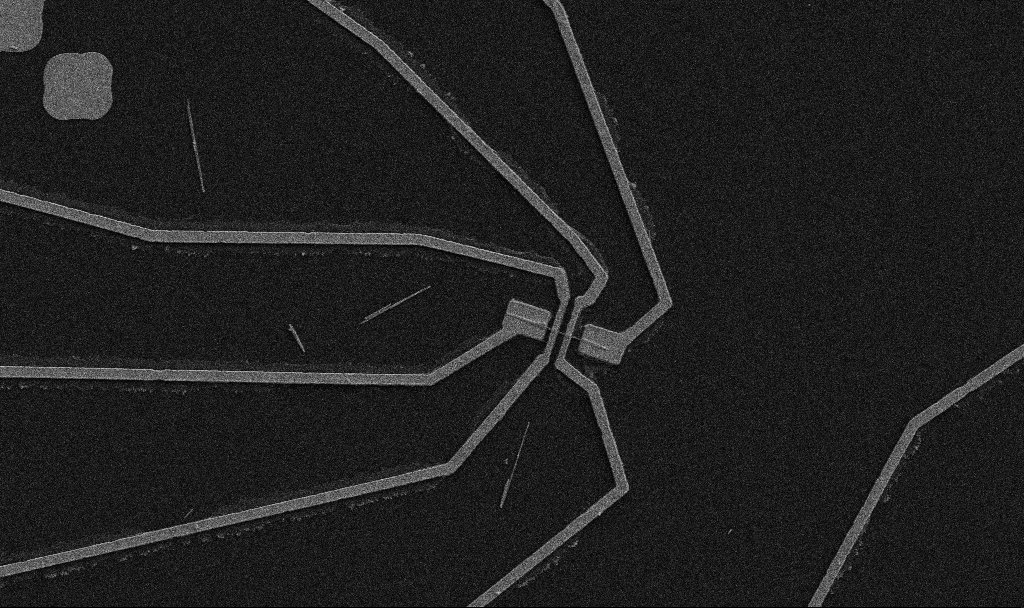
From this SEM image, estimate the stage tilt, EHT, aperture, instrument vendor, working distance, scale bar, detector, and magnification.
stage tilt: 0°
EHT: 5 kV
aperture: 30 µm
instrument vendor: Zeiss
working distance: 10.7 mm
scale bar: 10000 nm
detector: SE2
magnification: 5 K X